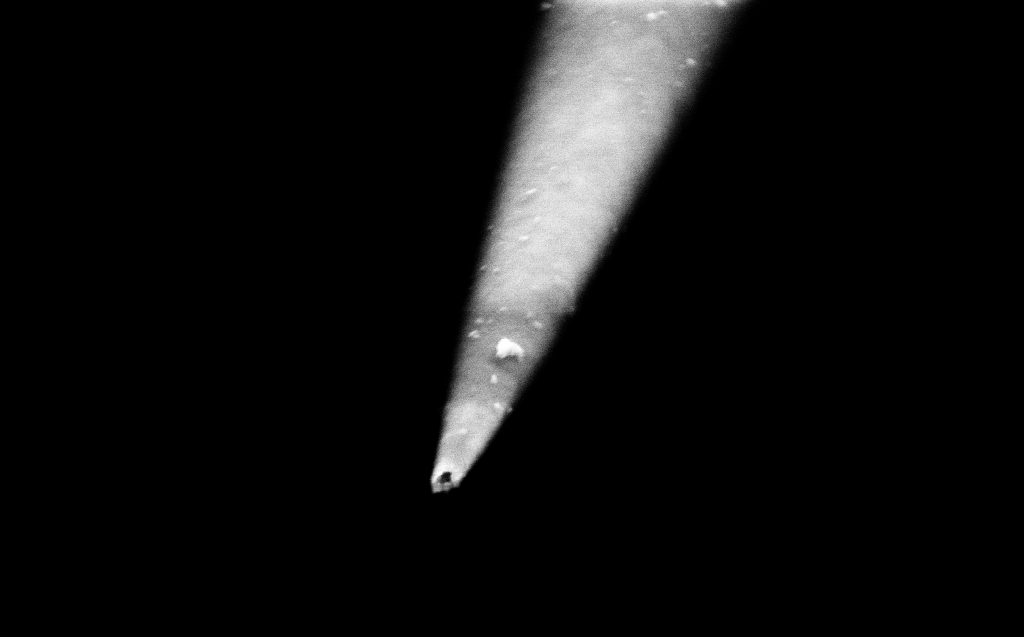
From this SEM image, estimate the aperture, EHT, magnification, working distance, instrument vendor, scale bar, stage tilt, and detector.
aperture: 30 µm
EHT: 2 kV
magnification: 100 K X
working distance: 4 mm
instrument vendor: Zeiss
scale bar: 200 nm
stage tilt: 45°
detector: InLens